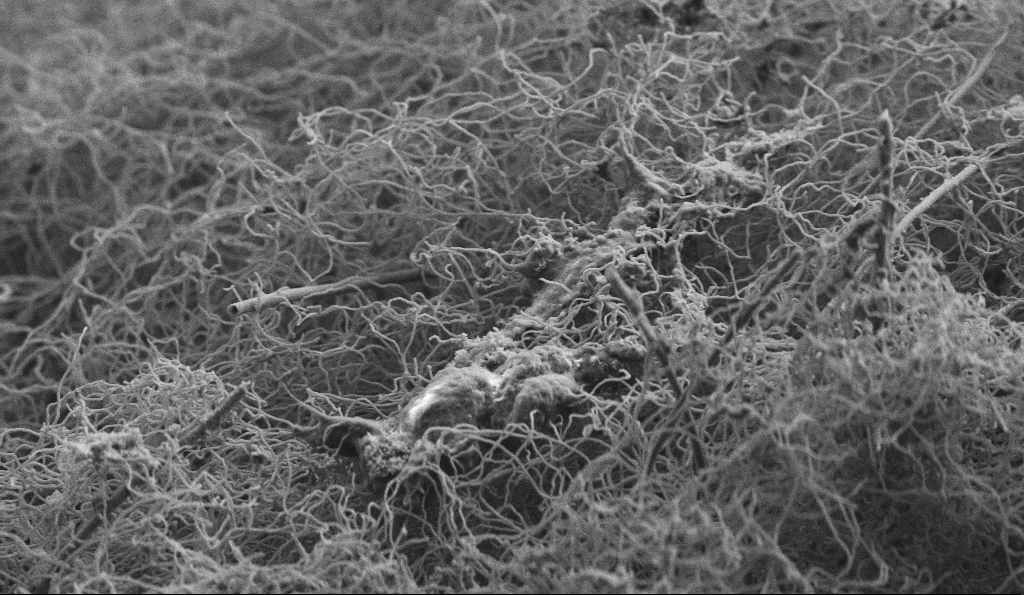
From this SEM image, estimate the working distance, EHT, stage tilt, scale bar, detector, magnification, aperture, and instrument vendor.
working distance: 4.6 mm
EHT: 3 kV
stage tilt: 0°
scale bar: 2000 nm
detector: SE2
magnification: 8 K X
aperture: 30 µm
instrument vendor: Zeiss